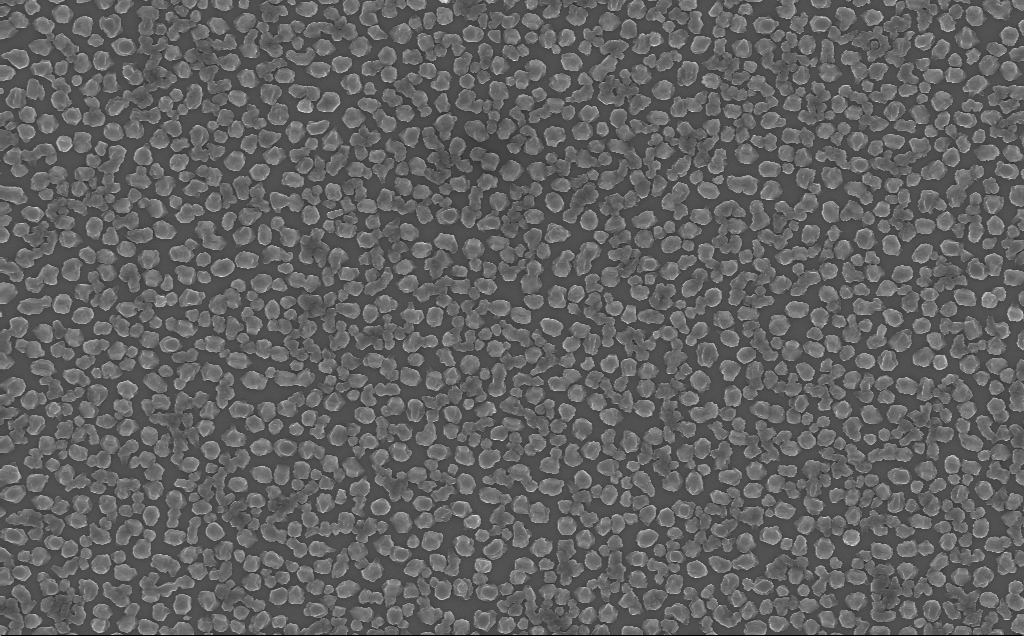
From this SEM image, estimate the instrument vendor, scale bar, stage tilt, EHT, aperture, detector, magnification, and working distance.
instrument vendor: Zeiss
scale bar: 2000 nm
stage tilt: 0°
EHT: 10 kV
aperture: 30 µm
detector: InLens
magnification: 11.85 K X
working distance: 7 mm